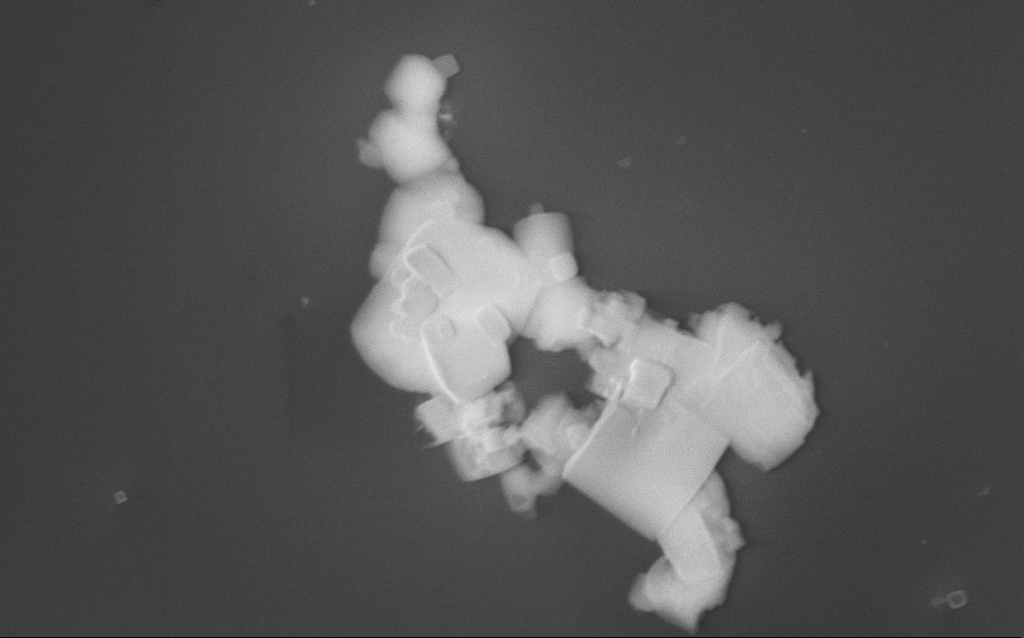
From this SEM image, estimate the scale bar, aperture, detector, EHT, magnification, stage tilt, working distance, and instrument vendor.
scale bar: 1000 nm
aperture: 30 µm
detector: InLens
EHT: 10 kV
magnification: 30.8 K X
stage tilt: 0°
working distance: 2 mm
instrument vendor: Zeiss